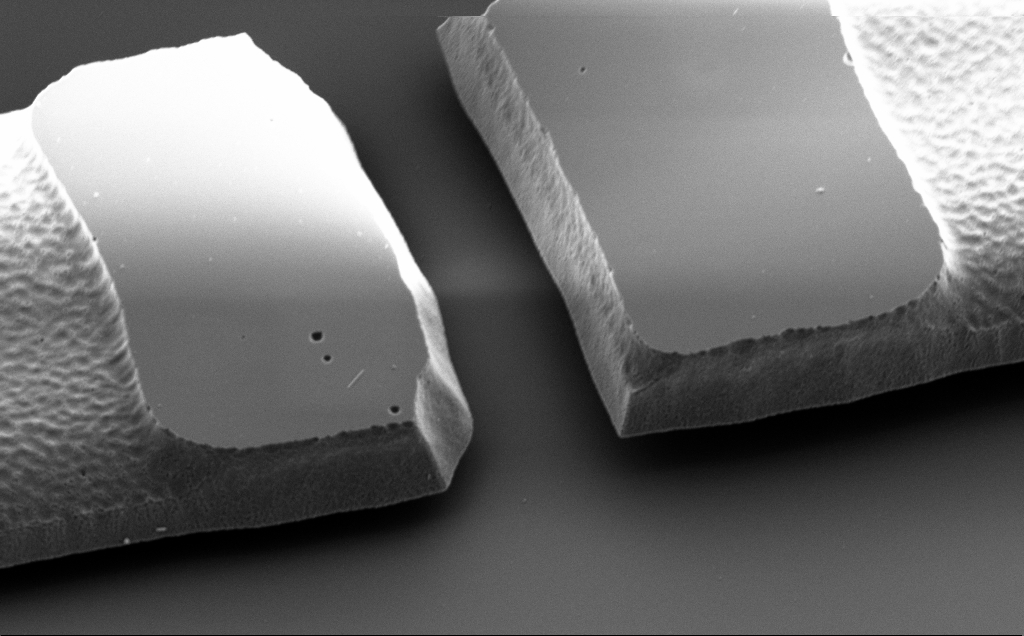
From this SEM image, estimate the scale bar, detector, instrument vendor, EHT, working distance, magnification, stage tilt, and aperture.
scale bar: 2000 nm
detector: SE2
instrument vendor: Zeiss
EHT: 2 kV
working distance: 10 mm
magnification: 12.75 K X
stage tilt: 43°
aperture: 30 µm